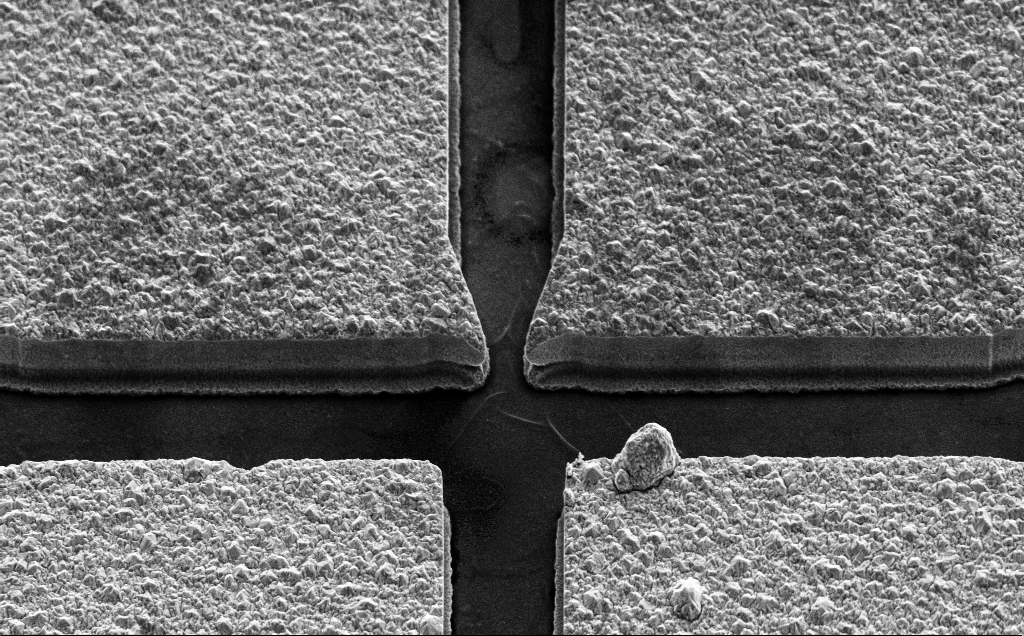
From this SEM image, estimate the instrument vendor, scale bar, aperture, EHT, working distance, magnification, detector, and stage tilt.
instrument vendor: Zeiss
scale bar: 10000 nm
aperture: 30 µm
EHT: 10 kV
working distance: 15 mm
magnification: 4.5 K X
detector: SE2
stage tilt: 45°